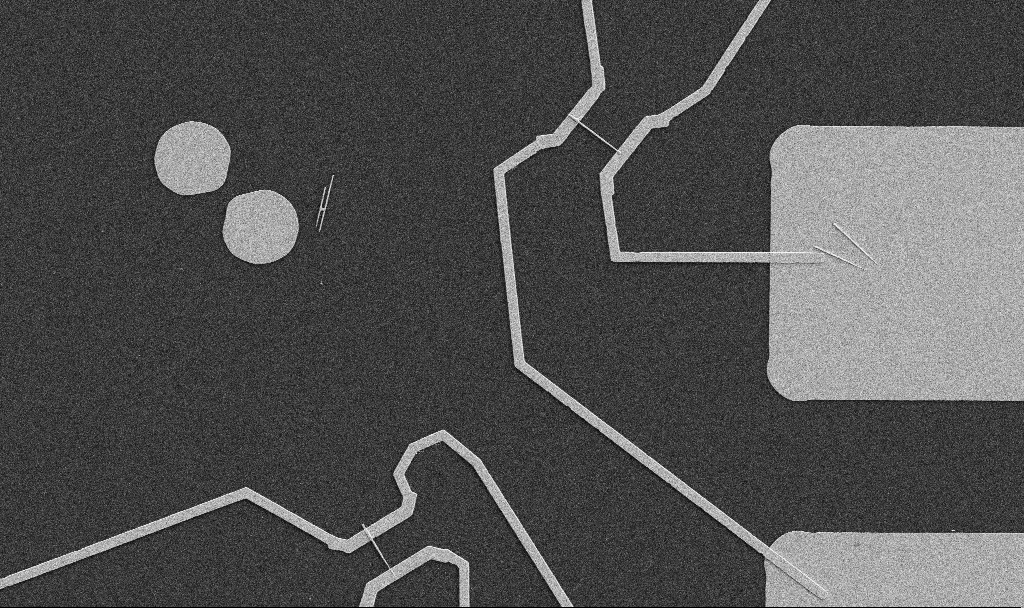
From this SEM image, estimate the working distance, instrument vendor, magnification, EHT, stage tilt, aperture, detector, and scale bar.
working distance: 10.7 mm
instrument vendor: Zeiss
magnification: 5 K X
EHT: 5 kV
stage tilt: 0°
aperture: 30 µm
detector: SE2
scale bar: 10000 nm